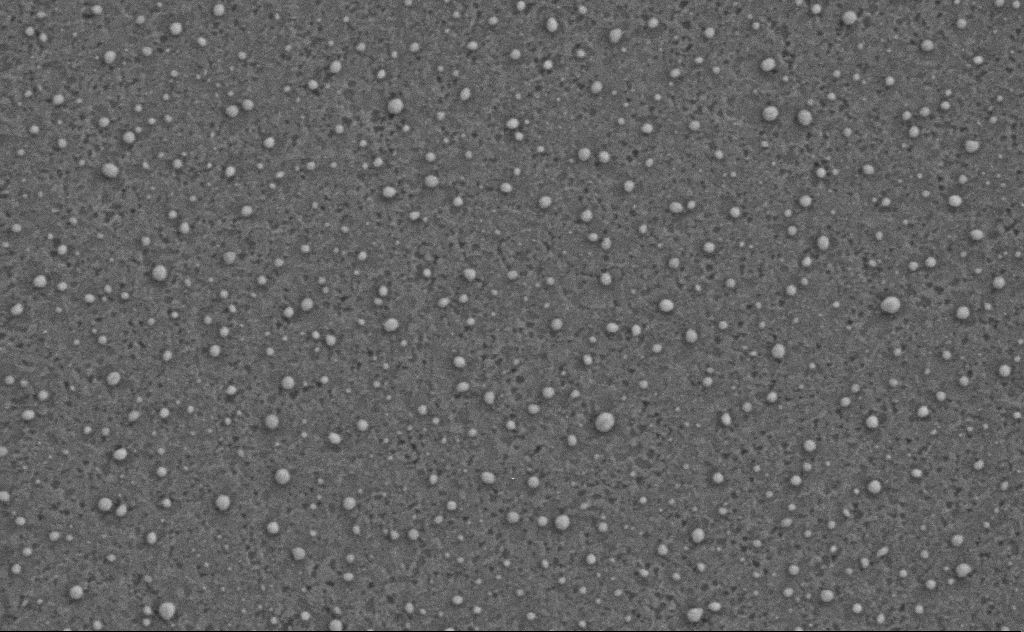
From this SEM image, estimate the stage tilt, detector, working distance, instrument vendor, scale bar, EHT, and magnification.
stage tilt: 0°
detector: SE2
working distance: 4 mm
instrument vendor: Zeiss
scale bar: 200 nm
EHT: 3 kV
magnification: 80 K X